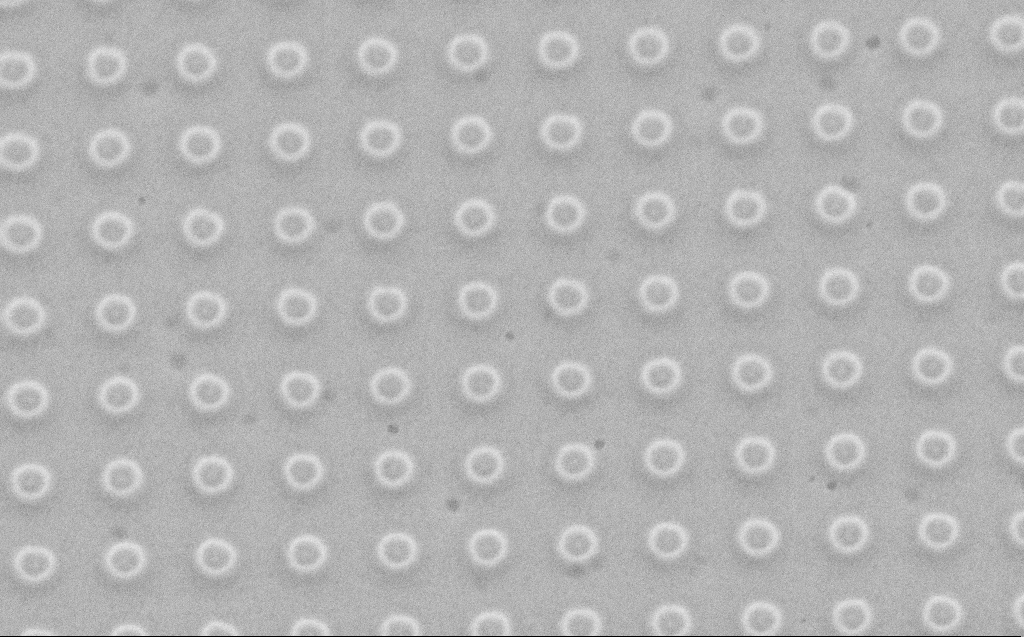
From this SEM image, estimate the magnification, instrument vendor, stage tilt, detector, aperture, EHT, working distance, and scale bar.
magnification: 42 K X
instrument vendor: Zeiss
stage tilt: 23.8°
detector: SE2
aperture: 30 µm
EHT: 5 kV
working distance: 4 mm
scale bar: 1000 nm